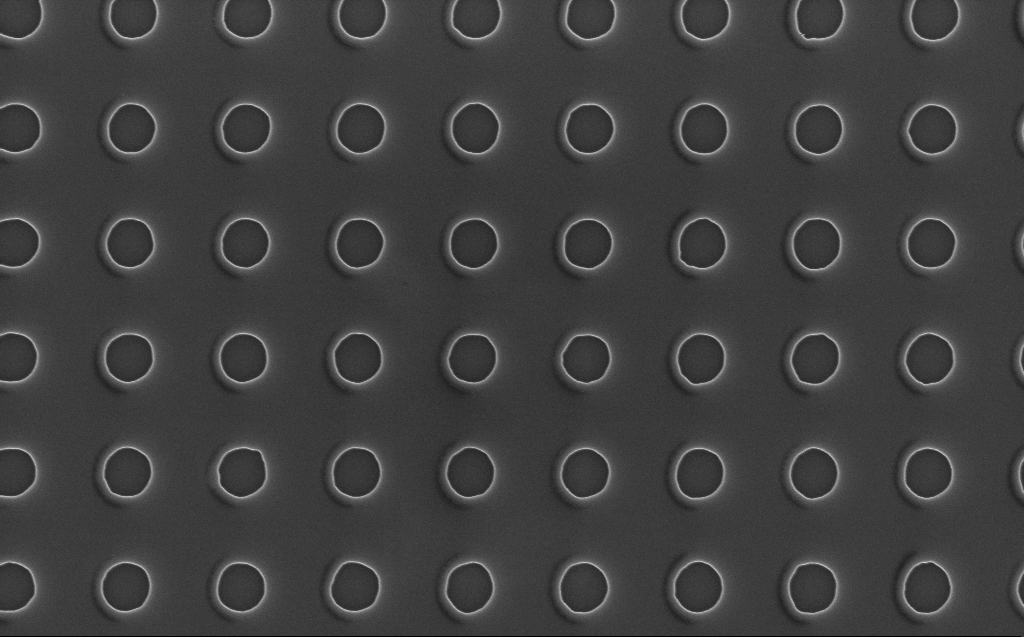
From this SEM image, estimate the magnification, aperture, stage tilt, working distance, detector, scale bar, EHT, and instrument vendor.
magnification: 40 K X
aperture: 30 µm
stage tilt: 0°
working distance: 7 mm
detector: InLens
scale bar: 1000 nm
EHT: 5 kV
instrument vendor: Zeiss